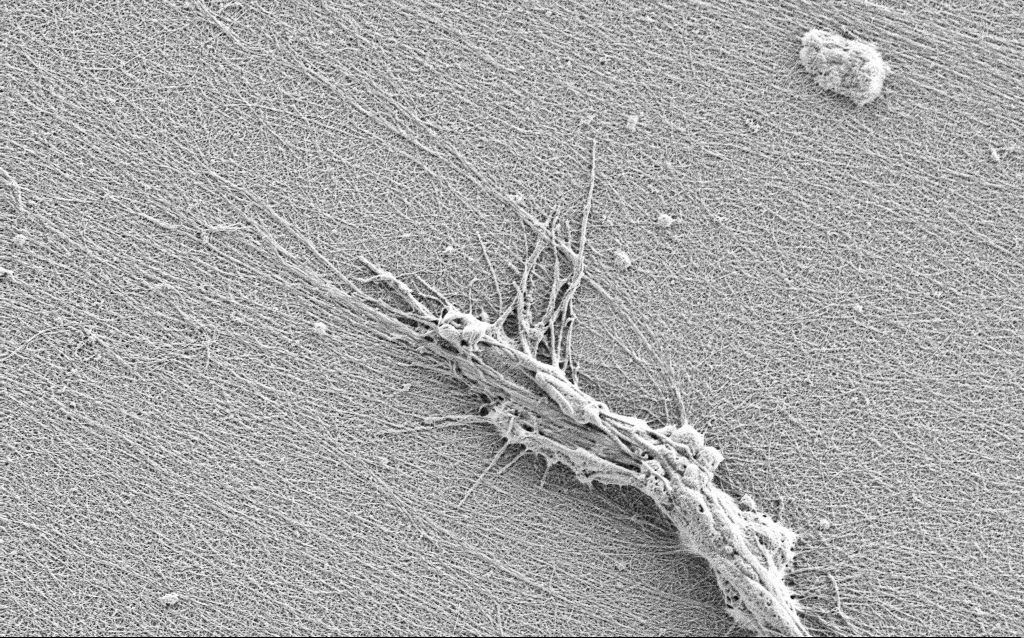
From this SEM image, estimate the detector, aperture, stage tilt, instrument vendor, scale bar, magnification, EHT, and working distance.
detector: SE2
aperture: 30 µm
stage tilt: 0°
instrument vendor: Zeiss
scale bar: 2000 nm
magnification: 10 K X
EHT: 0.9 kV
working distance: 3 mm